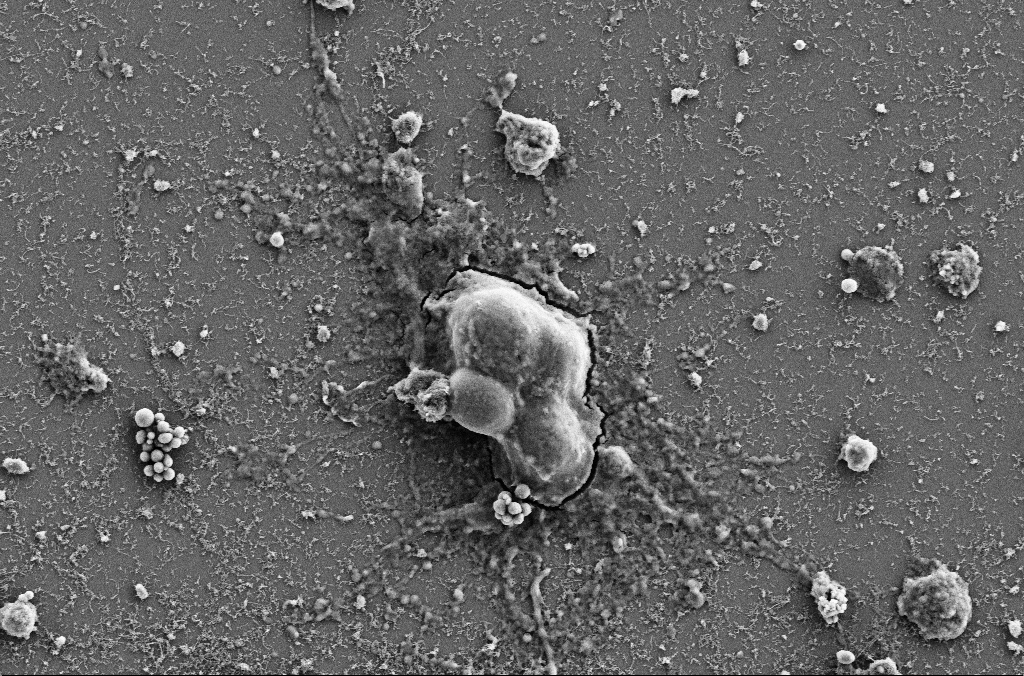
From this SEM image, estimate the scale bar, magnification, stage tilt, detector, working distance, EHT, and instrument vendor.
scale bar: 10000 nm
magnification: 5 K X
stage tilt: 0°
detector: SE2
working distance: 4 mm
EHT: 5 kV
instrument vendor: Zeiss